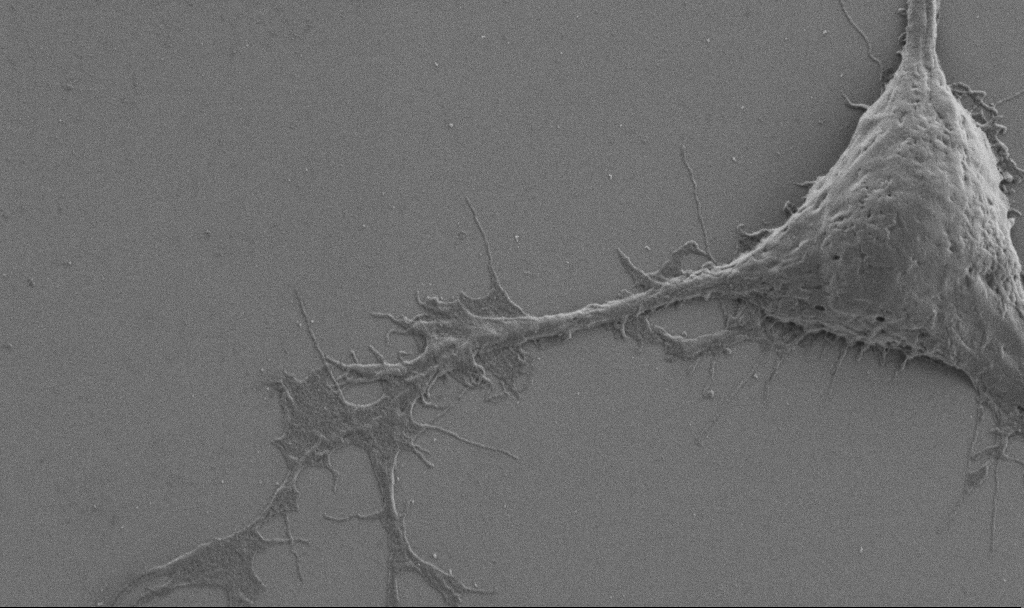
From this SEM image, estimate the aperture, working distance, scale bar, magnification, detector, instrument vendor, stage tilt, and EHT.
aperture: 30 µm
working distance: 6.9 mm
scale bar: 2000 nm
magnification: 10 K X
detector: SE2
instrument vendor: Zeiss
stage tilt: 0°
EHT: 1 kV